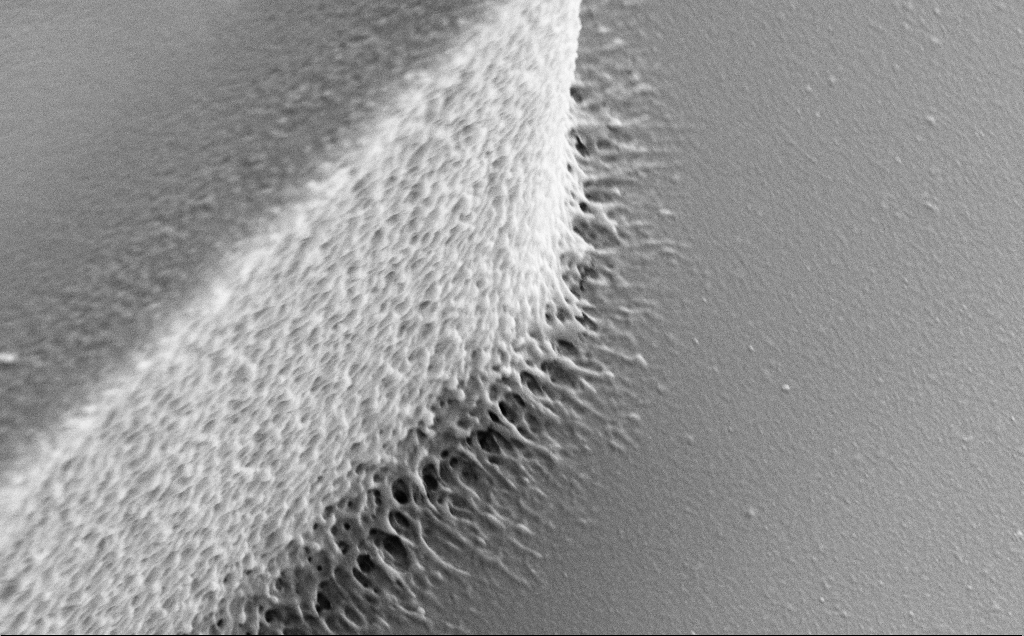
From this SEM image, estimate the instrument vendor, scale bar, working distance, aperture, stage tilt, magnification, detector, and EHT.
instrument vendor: Zeiss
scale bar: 1000 nm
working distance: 8 mm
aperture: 30 µm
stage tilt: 30°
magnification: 36.64 K X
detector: SE2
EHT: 5 kV